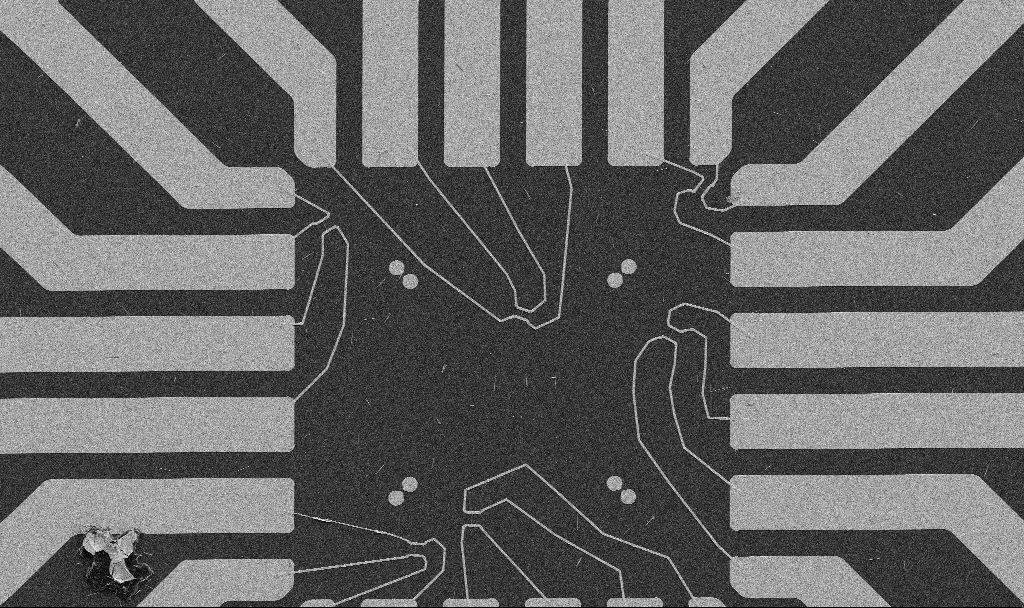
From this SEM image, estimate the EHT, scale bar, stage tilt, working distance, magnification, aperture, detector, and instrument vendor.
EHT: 5 kV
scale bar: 20000 nm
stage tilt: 0°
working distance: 10.7 mm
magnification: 1 K X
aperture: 30 µm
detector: SE2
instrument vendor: Zeiss